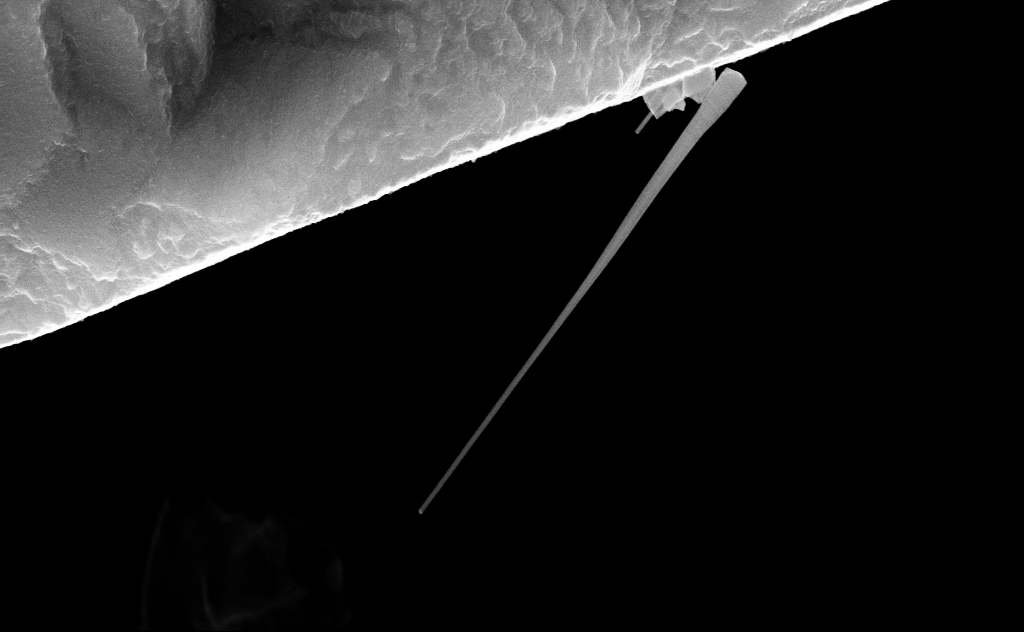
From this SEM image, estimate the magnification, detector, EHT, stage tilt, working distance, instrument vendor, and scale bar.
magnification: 57.06 K X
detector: InLens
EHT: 20 kV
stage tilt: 0°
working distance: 8 mm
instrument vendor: Zeiss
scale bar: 1000 nm